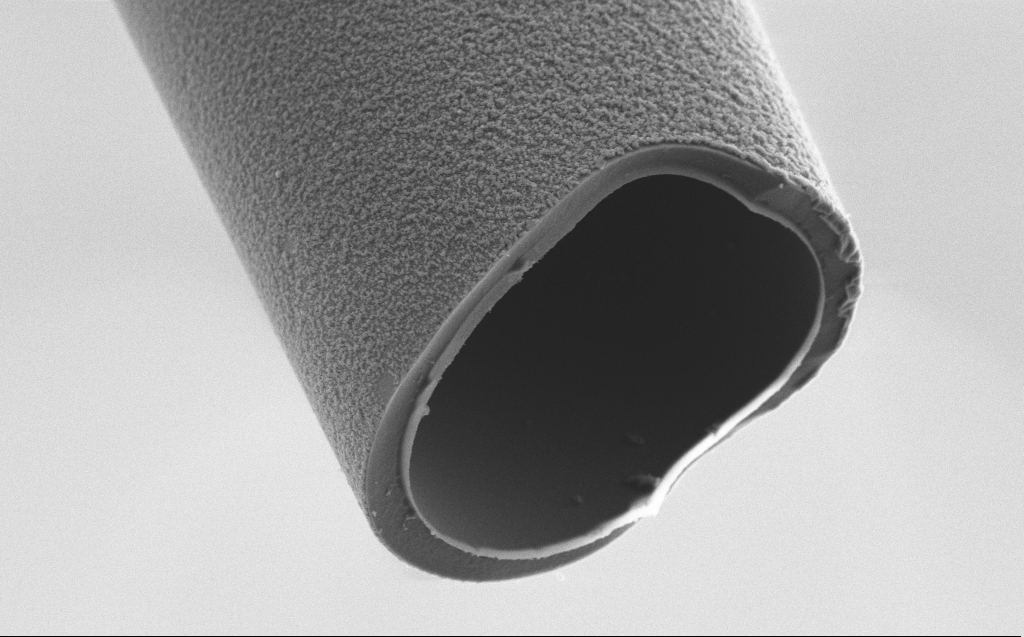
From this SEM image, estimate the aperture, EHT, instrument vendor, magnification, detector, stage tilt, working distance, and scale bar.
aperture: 30 µm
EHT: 5 kV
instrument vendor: Zeiss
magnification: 6.71 K X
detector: SE2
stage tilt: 45°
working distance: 4 mm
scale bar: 10000 nm